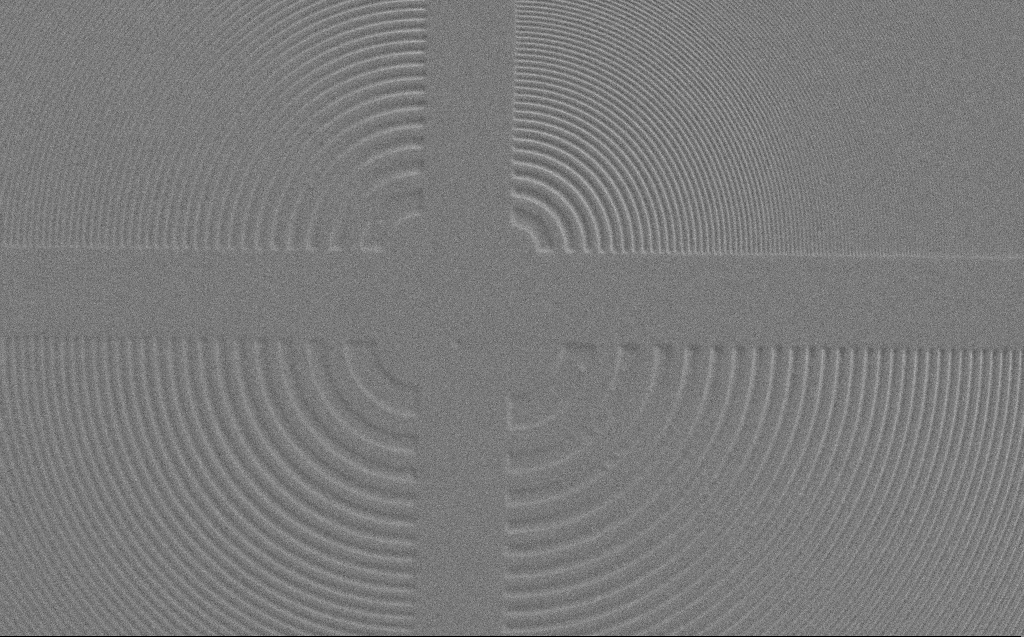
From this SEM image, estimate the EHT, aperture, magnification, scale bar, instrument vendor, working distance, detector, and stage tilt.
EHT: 3 kV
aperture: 30 µm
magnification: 3.33 K X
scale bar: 10000 nm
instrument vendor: Zeiss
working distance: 3 mm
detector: SE2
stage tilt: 0°